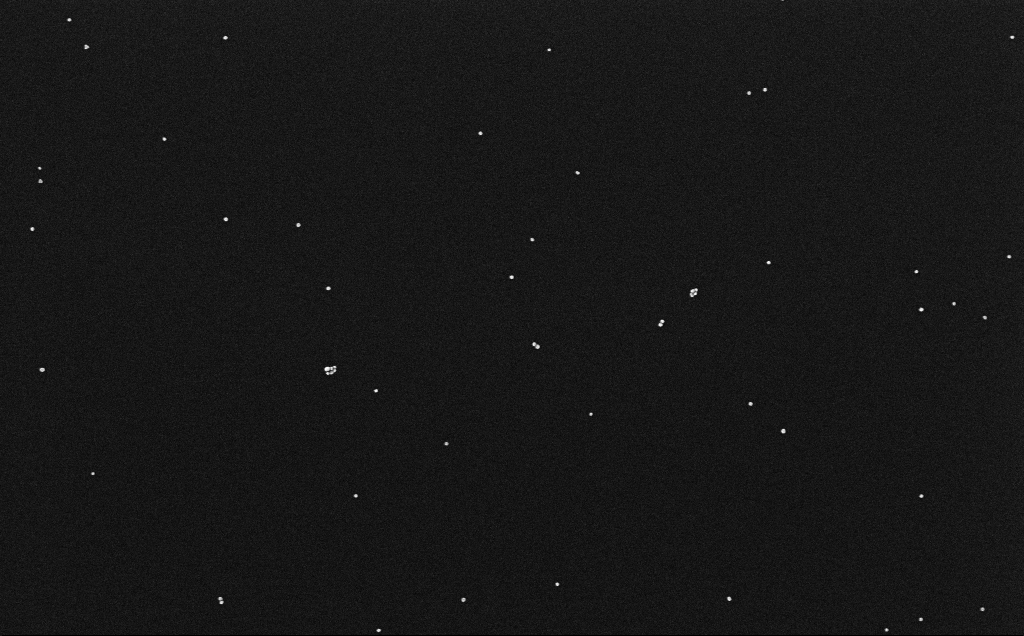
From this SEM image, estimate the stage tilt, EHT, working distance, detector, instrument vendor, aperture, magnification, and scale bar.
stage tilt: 0°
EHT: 10 kV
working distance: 3.2 mm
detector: InLens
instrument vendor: Zeiss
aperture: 30 µm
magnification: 100 K X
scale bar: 200 nm